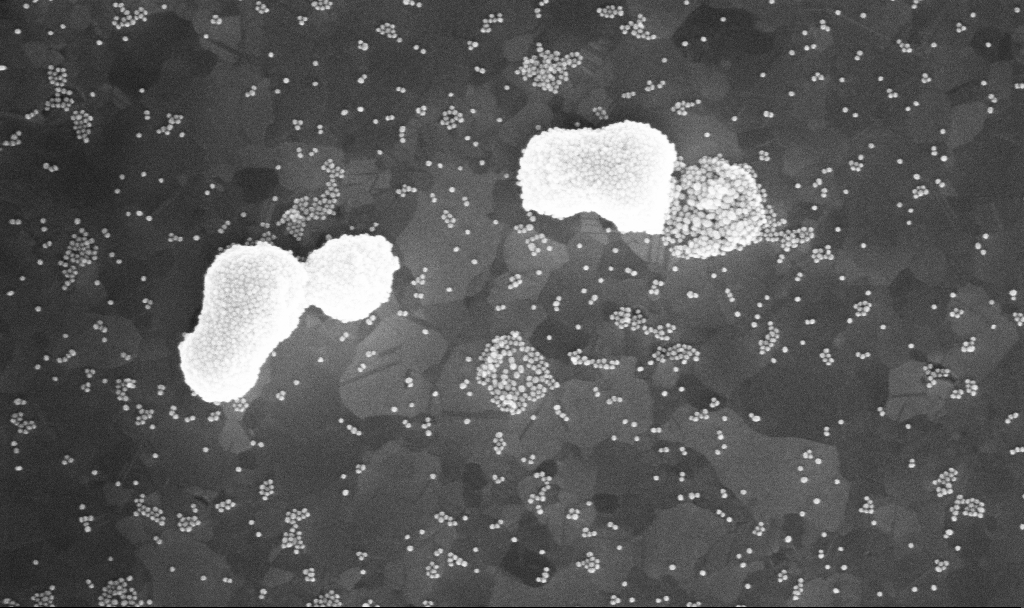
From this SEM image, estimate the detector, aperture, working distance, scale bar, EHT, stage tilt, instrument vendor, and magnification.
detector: InLens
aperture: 30 µm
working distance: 3.9 mm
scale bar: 200 nm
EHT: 10 kV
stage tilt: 0°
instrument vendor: Zeiss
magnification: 115.58 K X